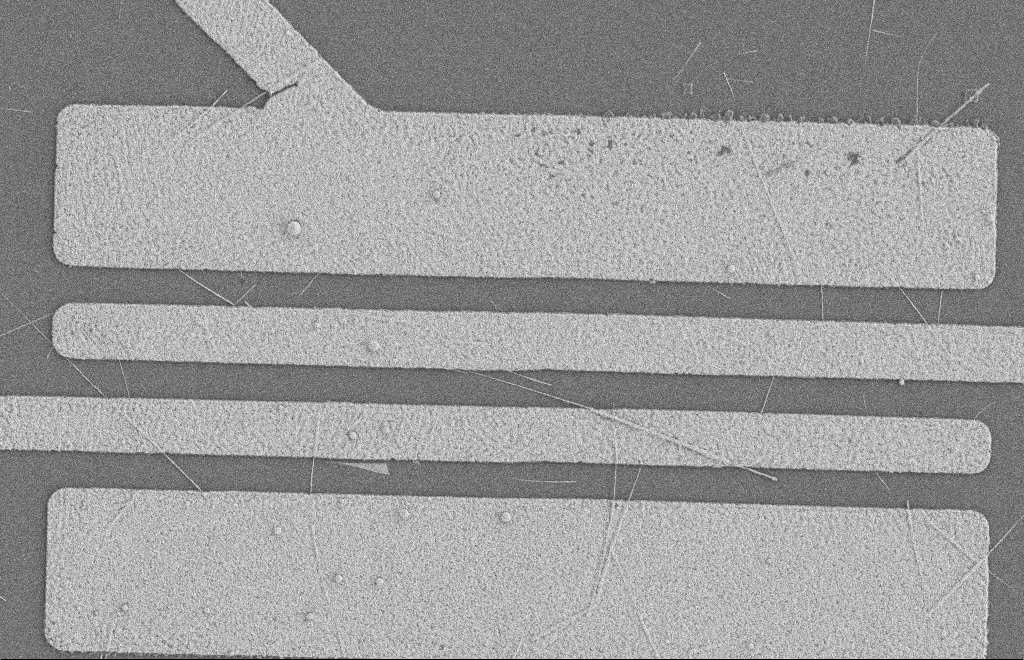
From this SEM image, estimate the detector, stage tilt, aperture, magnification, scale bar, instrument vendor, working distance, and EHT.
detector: SE2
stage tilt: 0°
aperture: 20 µm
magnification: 5.65 K X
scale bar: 2000 nm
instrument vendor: Zeiss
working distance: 8 mm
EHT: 2 kV